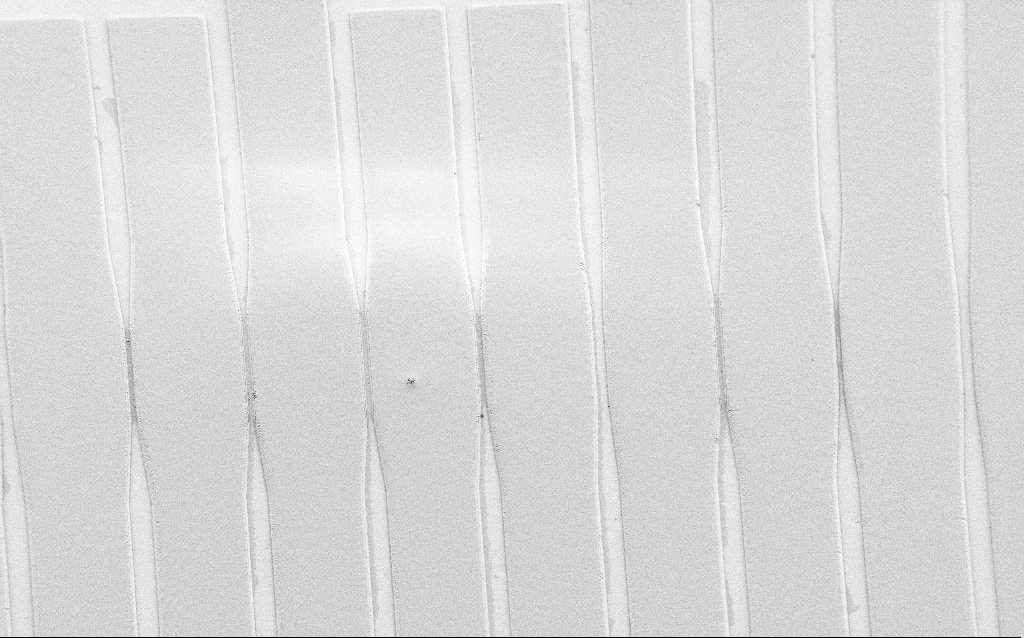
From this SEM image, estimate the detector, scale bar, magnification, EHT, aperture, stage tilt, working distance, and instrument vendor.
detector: SE2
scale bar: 100000 nm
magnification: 0.407 K X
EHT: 1 kV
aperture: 30 µm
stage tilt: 36°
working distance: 6 mm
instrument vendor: Zeiss